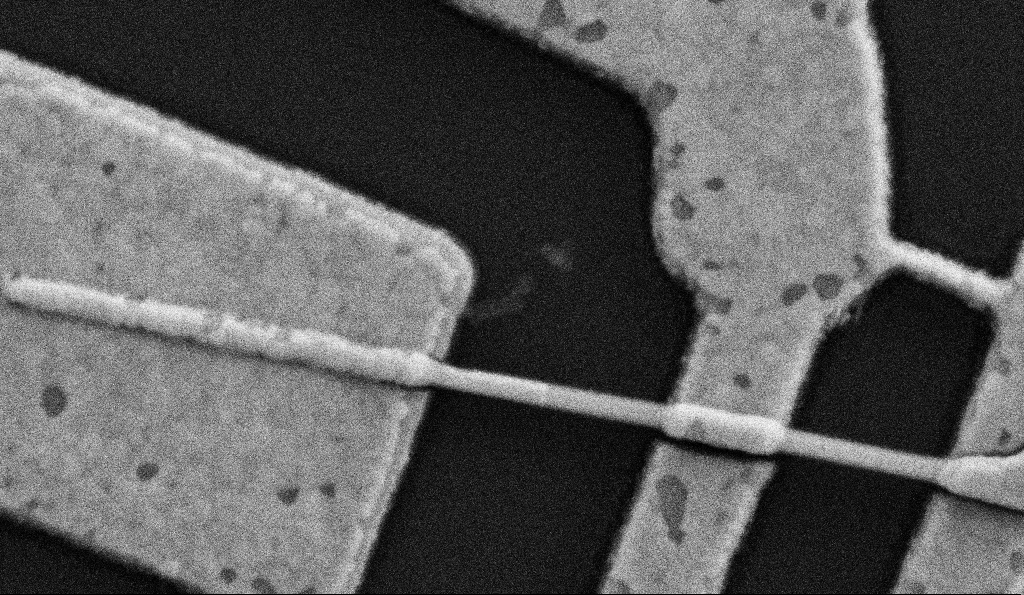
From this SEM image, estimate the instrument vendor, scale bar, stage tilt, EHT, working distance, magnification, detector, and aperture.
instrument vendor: Zeiss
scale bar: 200 nm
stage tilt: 0°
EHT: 5 kV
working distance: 8.5 mm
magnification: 100 K X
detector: SE2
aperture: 30 µm